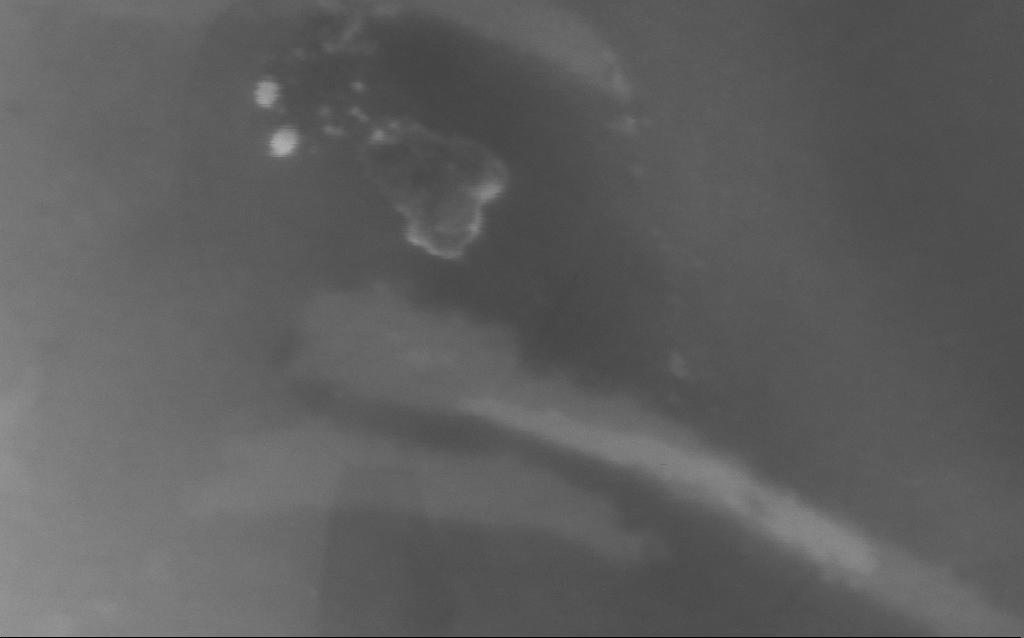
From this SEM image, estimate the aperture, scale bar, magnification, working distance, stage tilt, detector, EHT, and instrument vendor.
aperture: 30 µm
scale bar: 100 nm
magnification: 499.33 K X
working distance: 4 mm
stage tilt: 0°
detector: InLens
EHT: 5 kV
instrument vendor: Zeiss